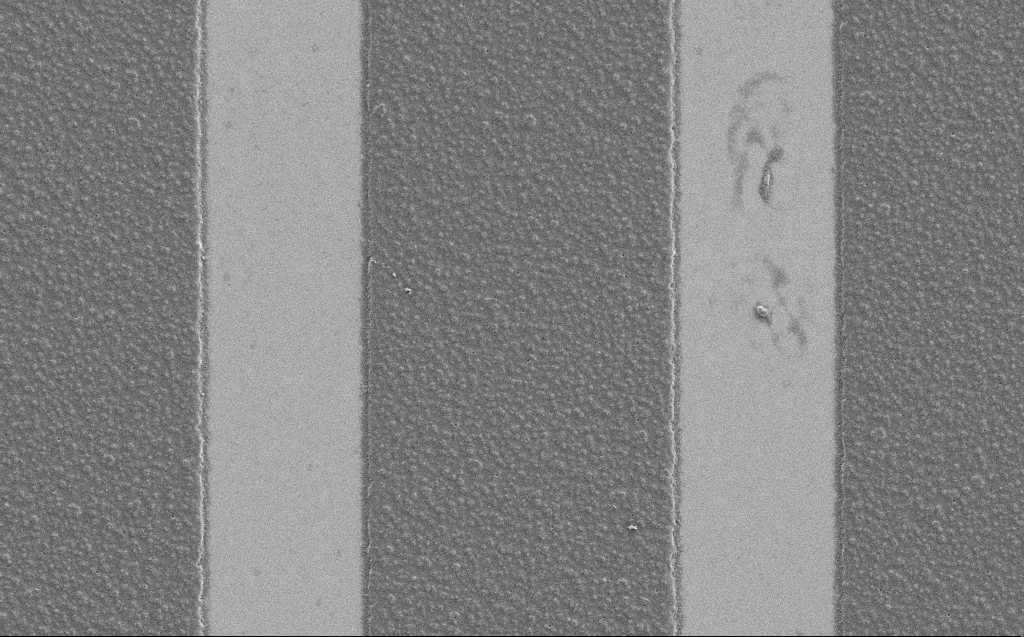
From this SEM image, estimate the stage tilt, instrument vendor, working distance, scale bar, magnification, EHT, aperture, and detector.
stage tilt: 0°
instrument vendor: Zeiss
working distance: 4 mm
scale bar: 10000 nm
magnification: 6.35 K X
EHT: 1.2 kV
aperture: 30 µm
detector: SE2